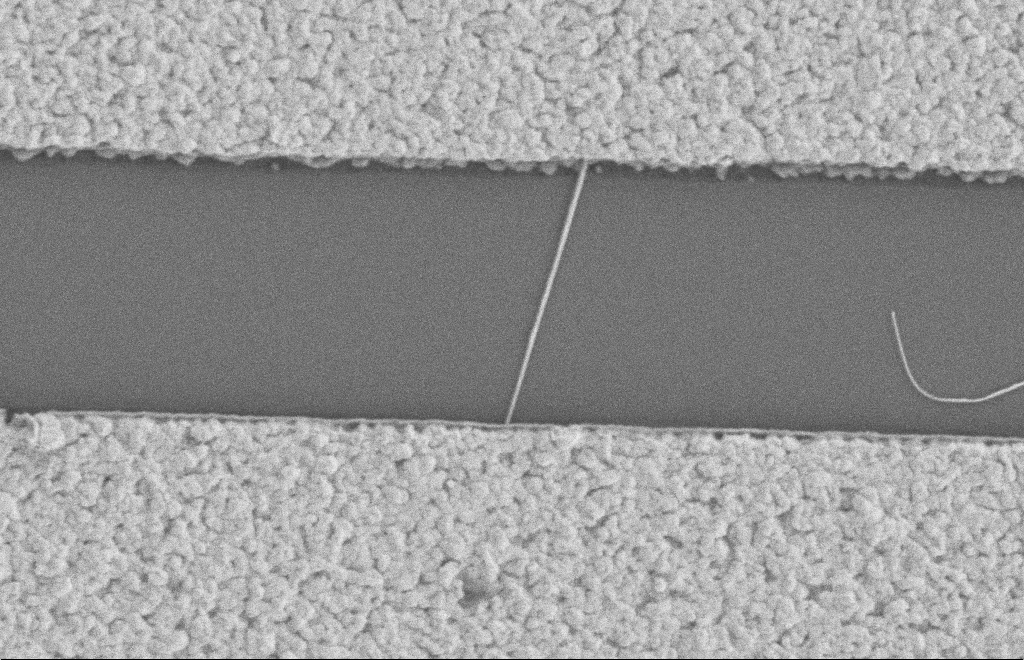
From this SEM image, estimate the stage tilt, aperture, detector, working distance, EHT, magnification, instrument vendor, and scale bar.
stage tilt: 0°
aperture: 20 µm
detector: SE2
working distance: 8 mm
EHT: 2 kV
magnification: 38.83 K X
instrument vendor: Zeiss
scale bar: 1000 nm